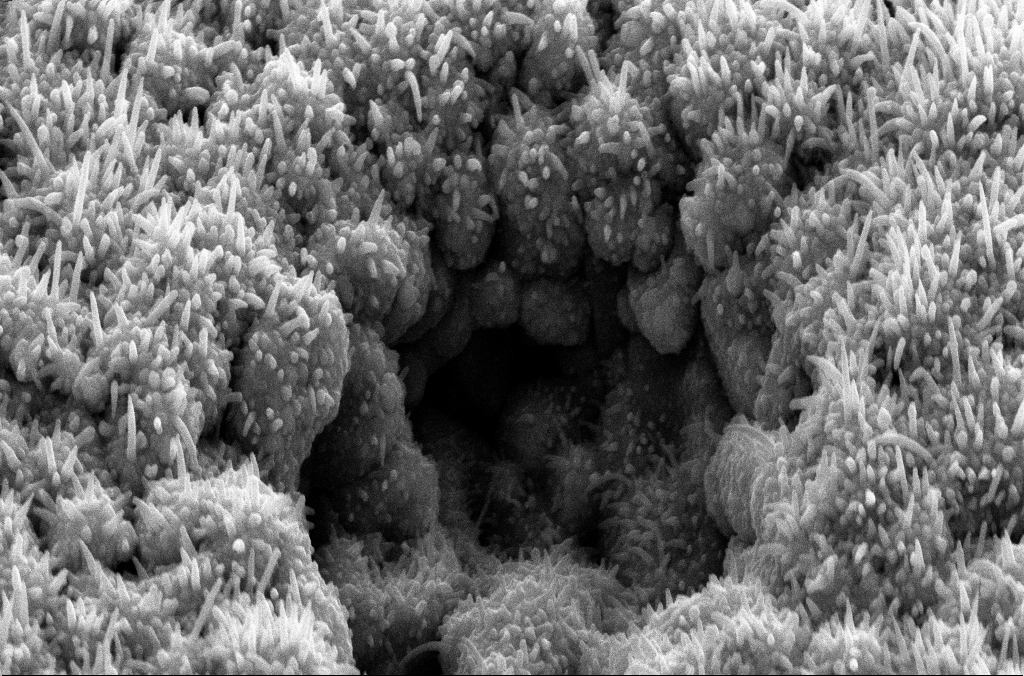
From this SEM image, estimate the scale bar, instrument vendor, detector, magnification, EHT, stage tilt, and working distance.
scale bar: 1000 nm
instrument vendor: Zeiss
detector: SE2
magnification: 44.05 K X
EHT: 10 kV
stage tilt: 45°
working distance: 8 mm